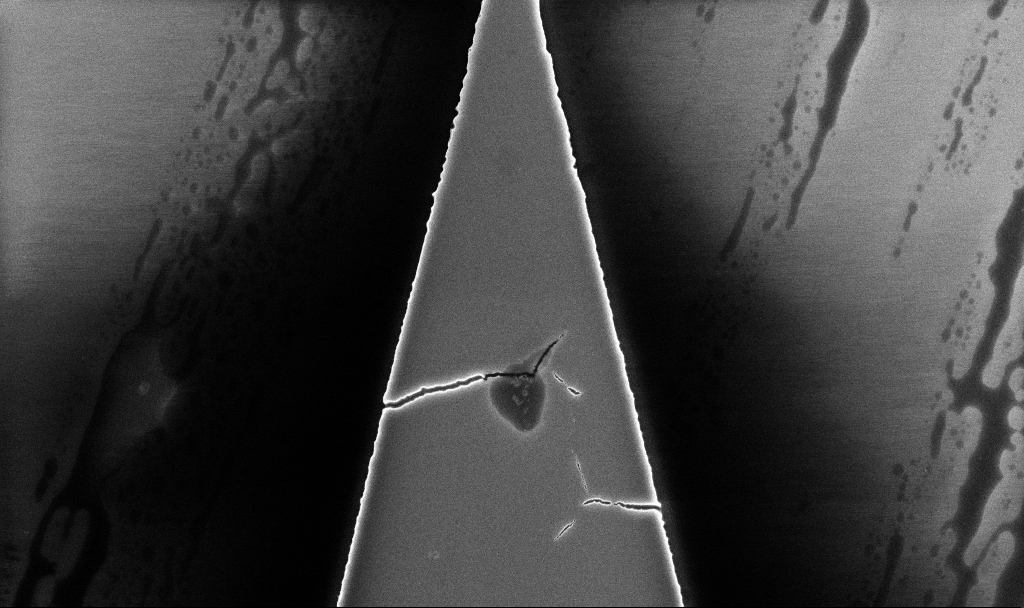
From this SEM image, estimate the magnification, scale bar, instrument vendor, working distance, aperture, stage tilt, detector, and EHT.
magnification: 15.72 K X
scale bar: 2000 nm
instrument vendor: Zeiss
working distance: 5.2 mm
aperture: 30 µm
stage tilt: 0°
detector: InLens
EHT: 5 kV